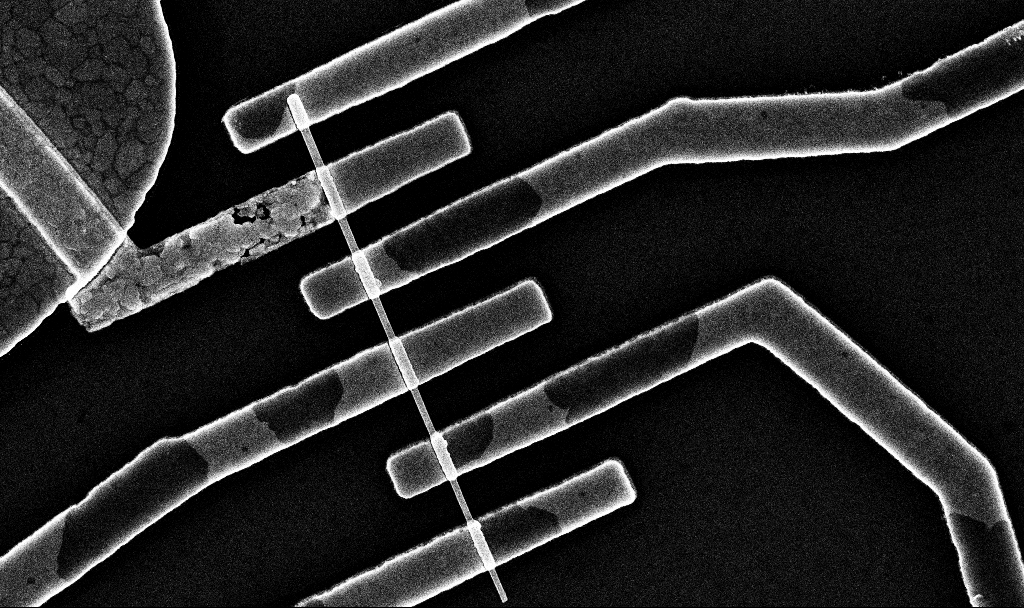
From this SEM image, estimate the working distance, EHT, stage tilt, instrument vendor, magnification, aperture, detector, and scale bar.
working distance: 6.7 mm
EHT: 10 kV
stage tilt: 0°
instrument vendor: Zeiss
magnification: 31.12 K X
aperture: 30 µm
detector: InLens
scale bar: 2000 nm